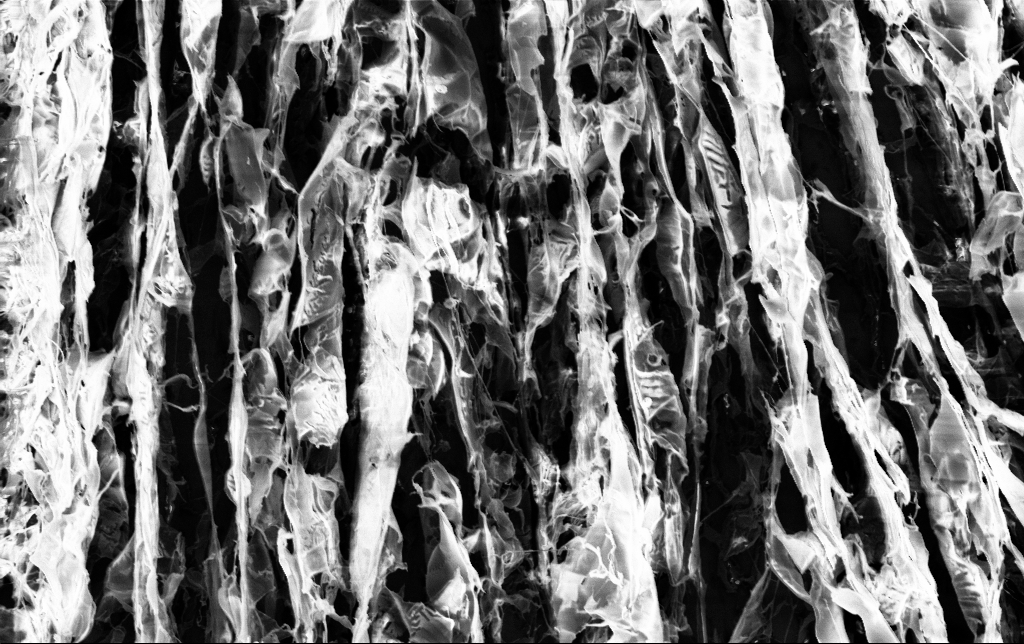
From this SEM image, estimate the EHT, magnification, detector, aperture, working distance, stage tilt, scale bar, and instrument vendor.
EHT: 3 kV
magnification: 5.12 K X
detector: InLens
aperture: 30 µm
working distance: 3.4 mm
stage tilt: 0°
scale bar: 10000 nm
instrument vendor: Zeiss